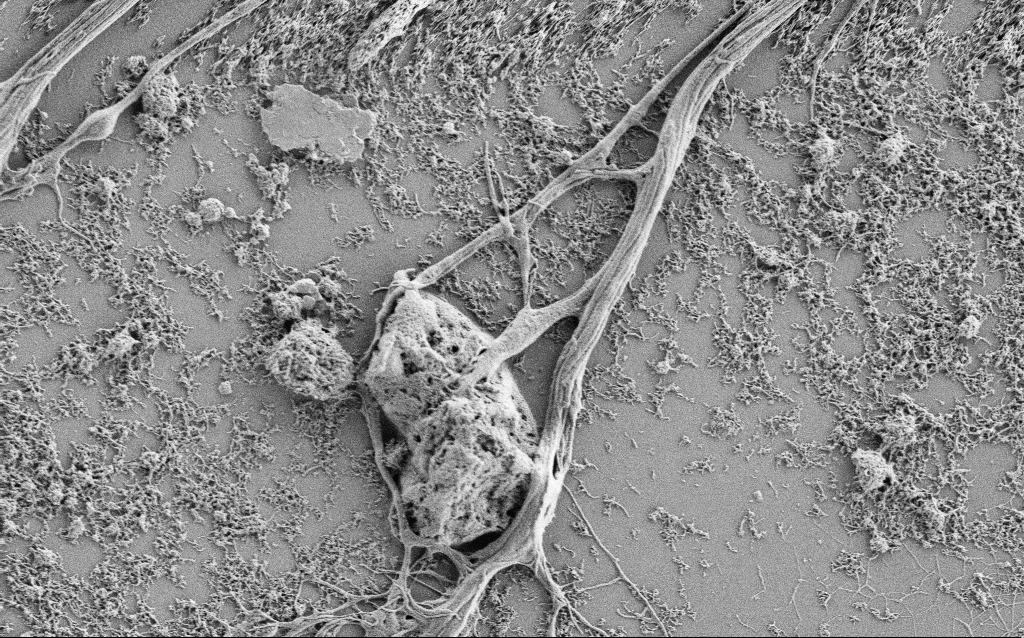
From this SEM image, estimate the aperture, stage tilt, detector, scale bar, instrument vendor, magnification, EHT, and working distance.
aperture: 30 µm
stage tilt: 0°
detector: SE2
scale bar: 2000 nm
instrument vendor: Zeiss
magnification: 7.5 K X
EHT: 1 kV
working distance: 4 mm